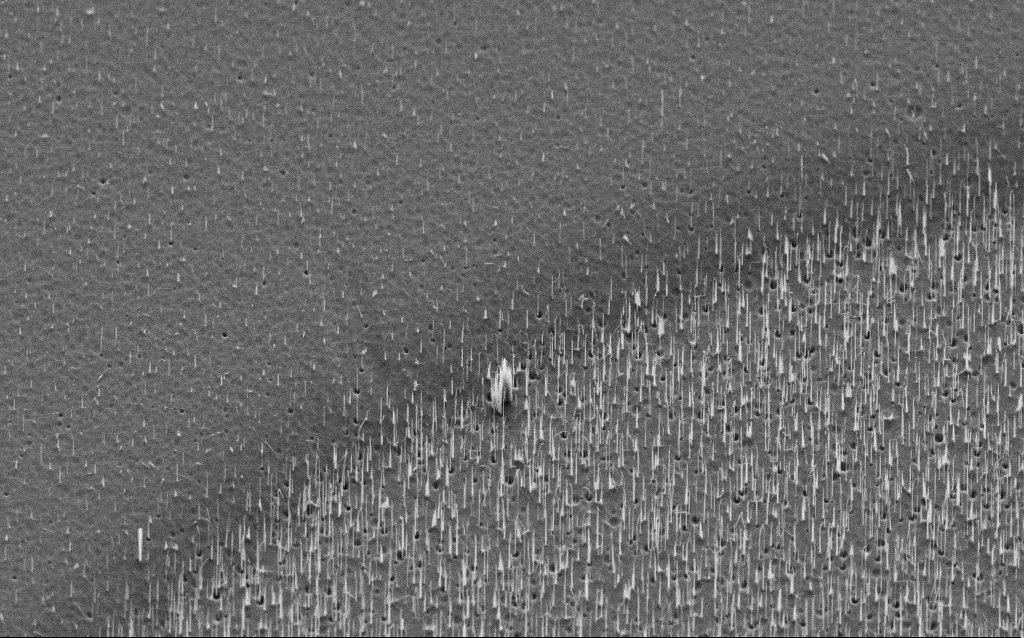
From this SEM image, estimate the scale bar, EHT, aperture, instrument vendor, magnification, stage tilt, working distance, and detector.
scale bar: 10000 nm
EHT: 5 kV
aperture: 30 µm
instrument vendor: Zeiss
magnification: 5.44 K X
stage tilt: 45°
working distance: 8 mm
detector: SE2